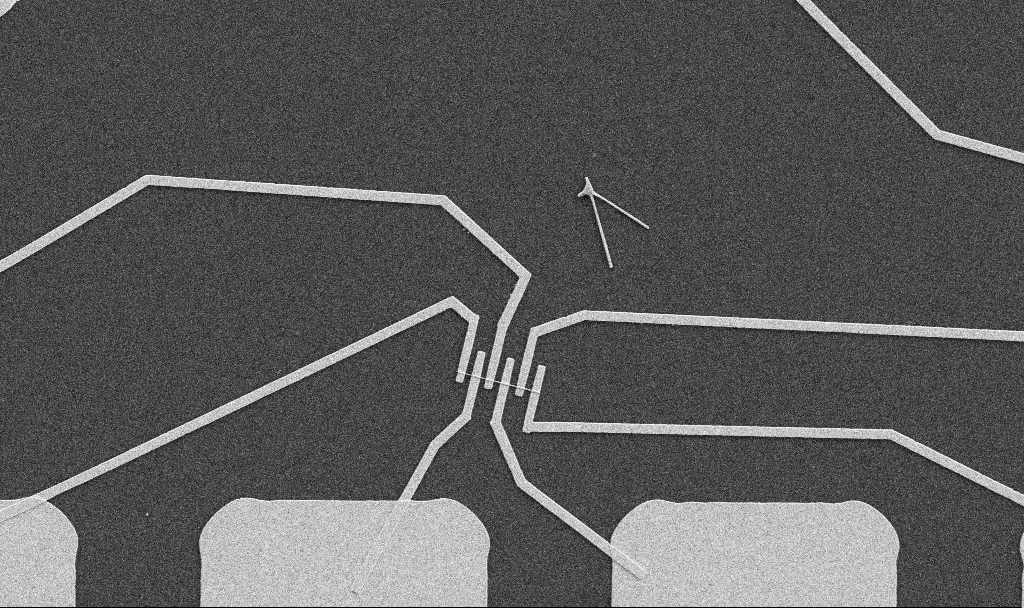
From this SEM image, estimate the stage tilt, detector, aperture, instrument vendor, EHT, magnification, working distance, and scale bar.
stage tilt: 0°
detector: SE2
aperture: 30 µm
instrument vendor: Zeiss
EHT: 5 kV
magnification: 5 K X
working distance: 10.7 mm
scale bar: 10000 nm